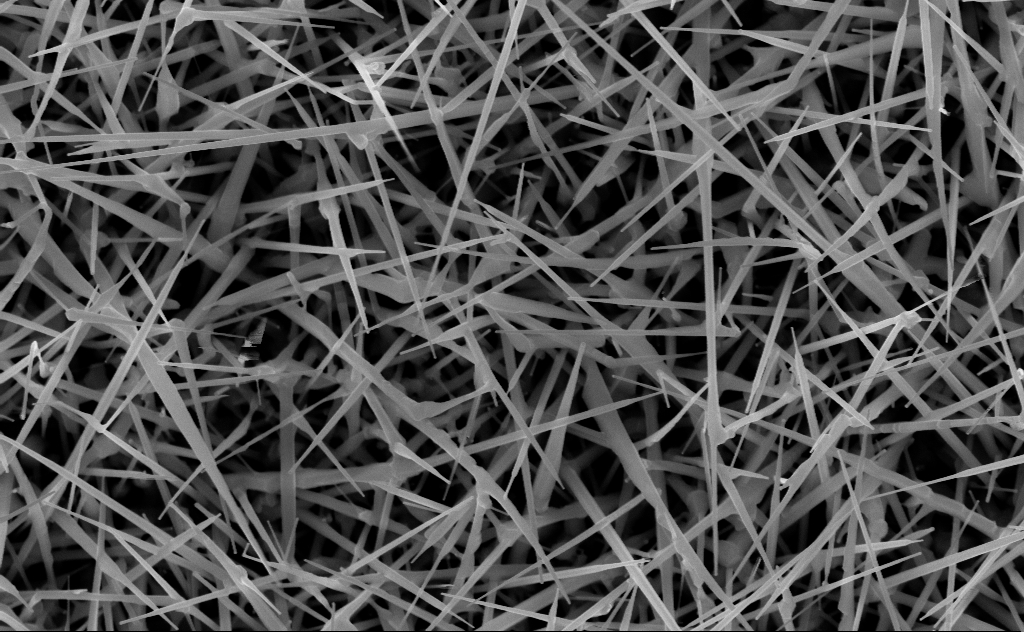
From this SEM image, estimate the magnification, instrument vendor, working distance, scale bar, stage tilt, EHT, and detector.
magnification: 40 K X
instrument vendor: Zeiss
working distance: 10 mm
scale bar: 1000 nm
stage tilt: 0°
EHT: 10 kV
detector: InLens